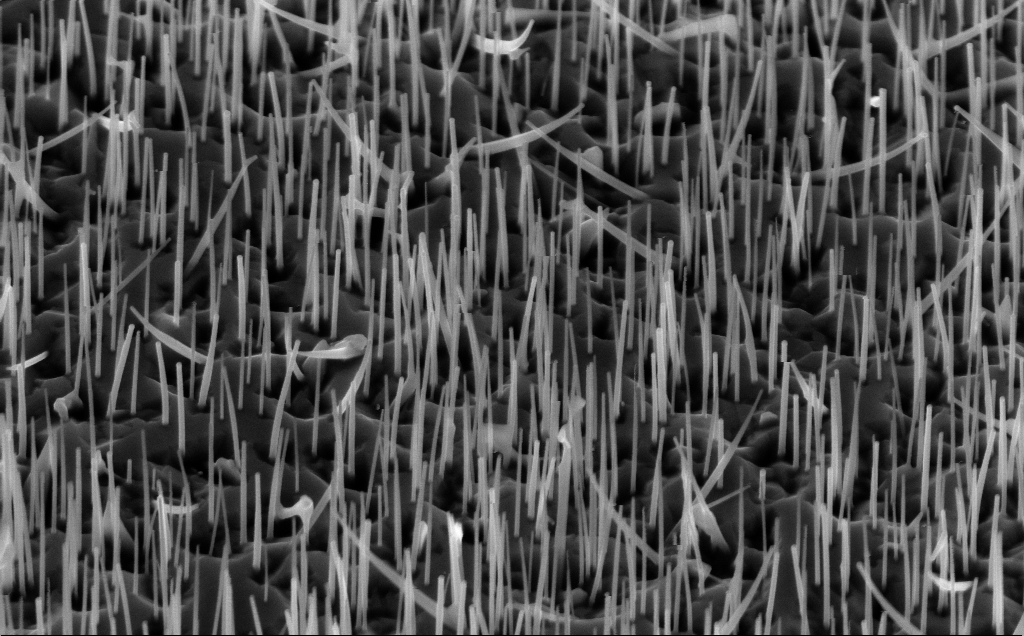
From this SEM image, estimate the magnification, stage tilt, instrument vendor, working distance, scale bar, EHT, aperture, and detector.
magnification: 40 K X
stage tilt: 45°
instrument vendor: Zeiss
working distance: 5 mm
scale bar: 1000 nm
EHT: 10 kV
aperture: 30 µm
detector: InLens